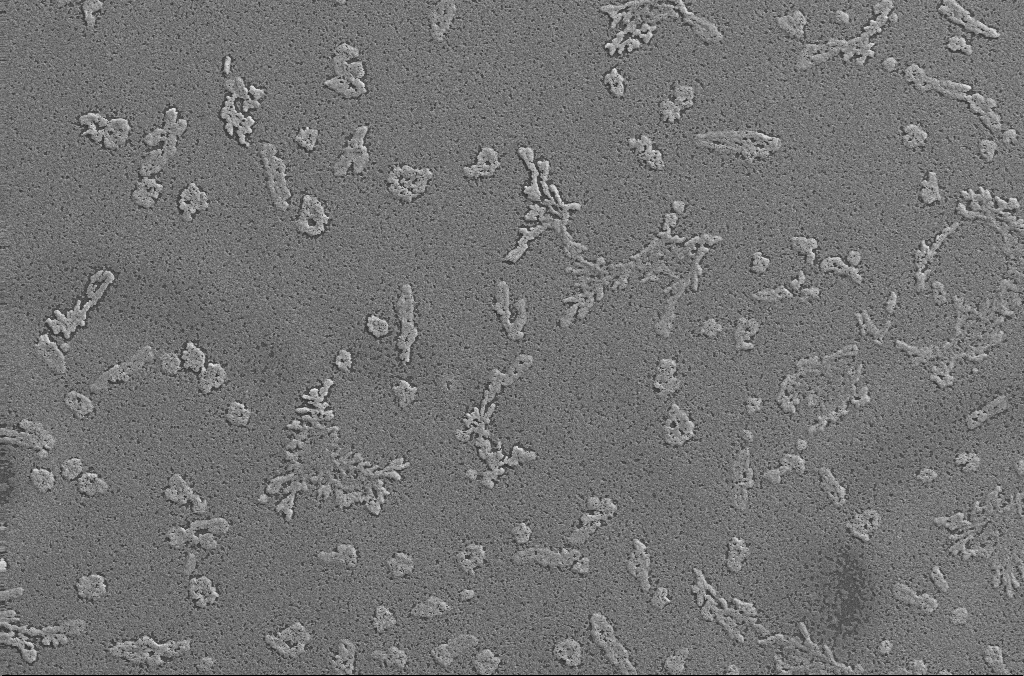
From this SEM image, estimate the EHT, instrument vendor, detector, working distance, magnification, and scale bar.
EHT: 20 kV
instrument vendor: Zeiss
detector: SE2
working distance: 2.9 mm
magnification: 0.5 K X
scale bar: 100000 nm